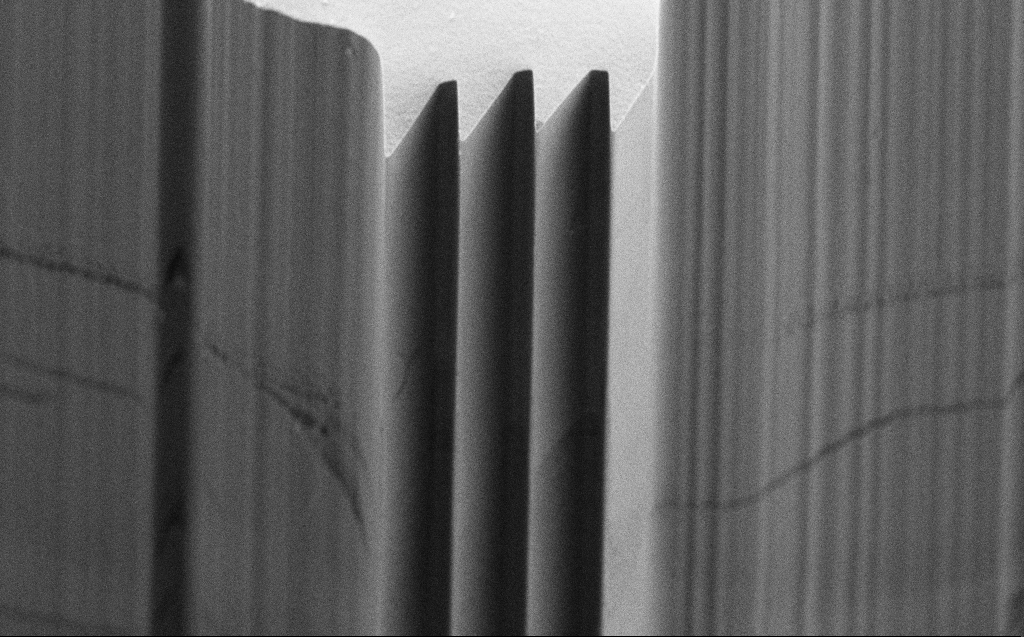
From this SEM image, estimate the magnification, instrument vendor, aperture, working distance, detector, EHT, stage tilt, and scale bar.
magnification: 2.44 K X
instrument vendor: Zeiss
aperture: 30 µm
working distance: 3 mm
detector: SE2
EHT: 10 kV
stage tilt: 45°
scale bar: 10000 nm